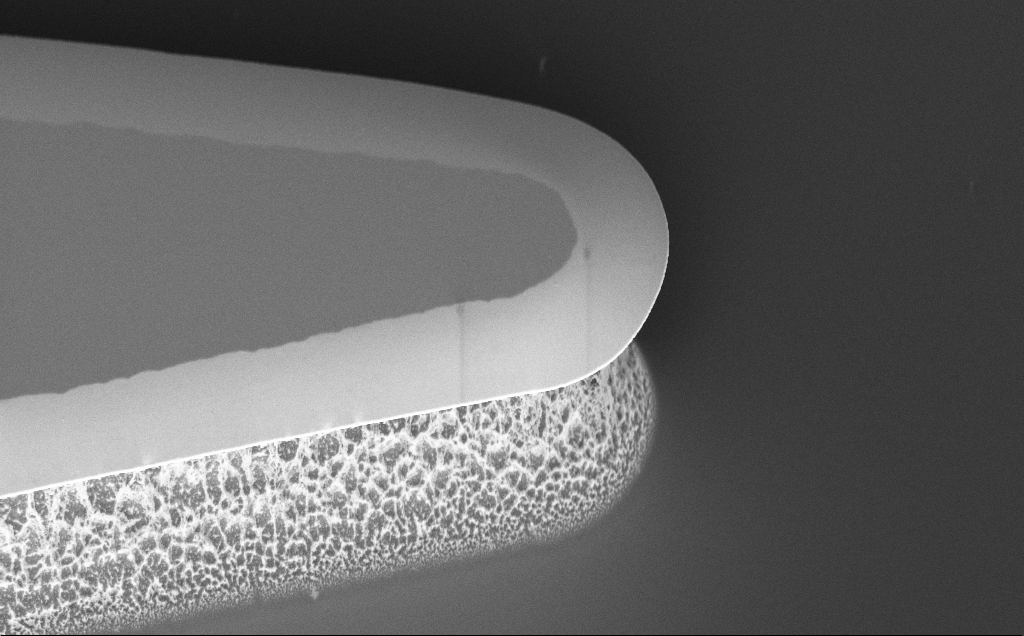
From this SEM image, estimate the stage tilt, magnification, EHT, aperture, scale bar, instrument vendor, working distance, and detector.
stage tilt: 45°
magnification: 5.38 K X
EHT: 5 kV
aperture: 30 µm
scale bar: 10000 nm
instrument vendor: Zeiss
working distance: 7 mm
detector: InLens